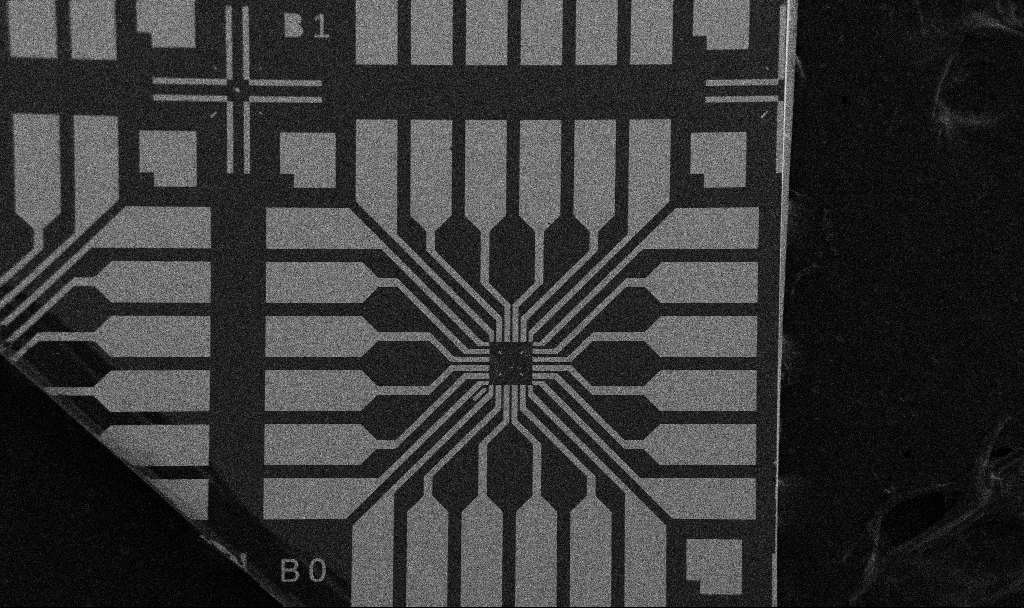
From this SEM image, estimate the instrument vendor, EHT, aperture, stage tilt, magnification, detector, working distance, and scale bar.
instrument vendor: Zeiss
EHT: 5 kV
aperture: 30 µm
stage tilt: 0°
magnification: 0.1 K X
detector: SE2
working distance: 10.7 mm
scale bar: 200000 nm